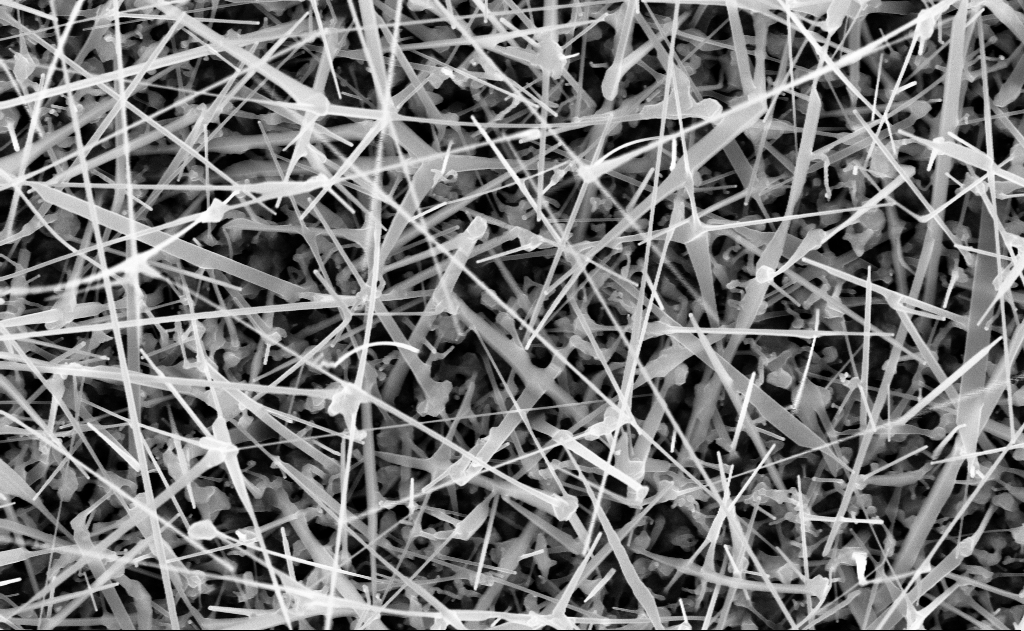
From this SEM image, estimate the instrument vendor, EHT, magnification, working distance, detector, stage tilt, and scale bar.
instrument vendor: Zeiss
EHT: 10 kV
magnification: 40 K X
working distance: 11 mm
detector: InLens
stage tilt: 0°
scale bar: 1000 nm